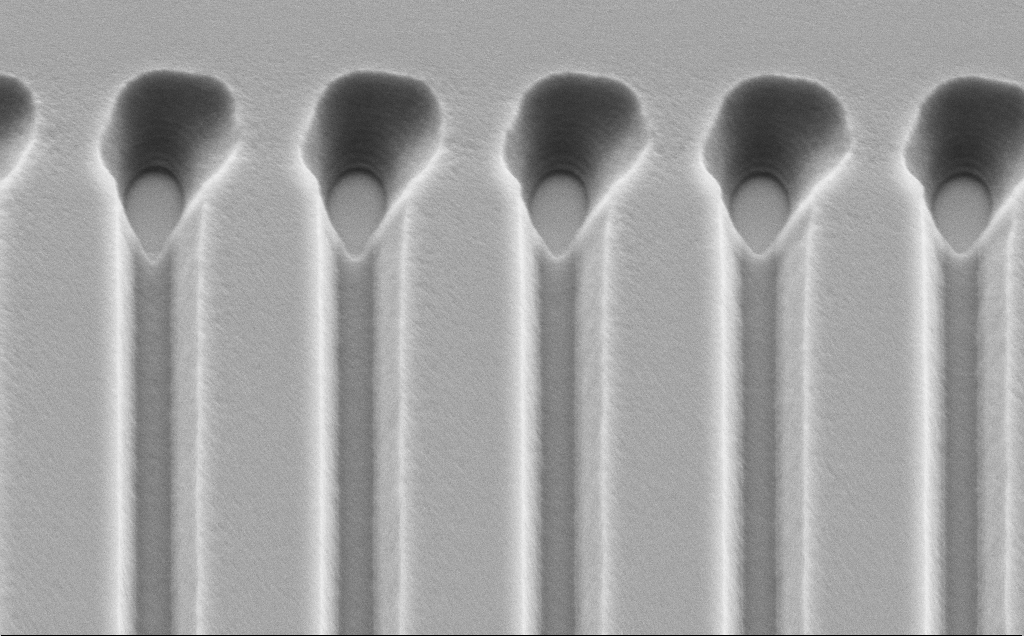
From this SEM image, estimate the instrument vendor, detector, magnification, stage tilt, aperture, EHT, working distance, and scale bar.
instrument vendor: Zeiss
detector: SE2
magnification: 18.42 K X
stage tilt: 45°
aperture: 30 µm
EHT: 5 kV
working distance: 10 mm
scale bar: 2000 nm